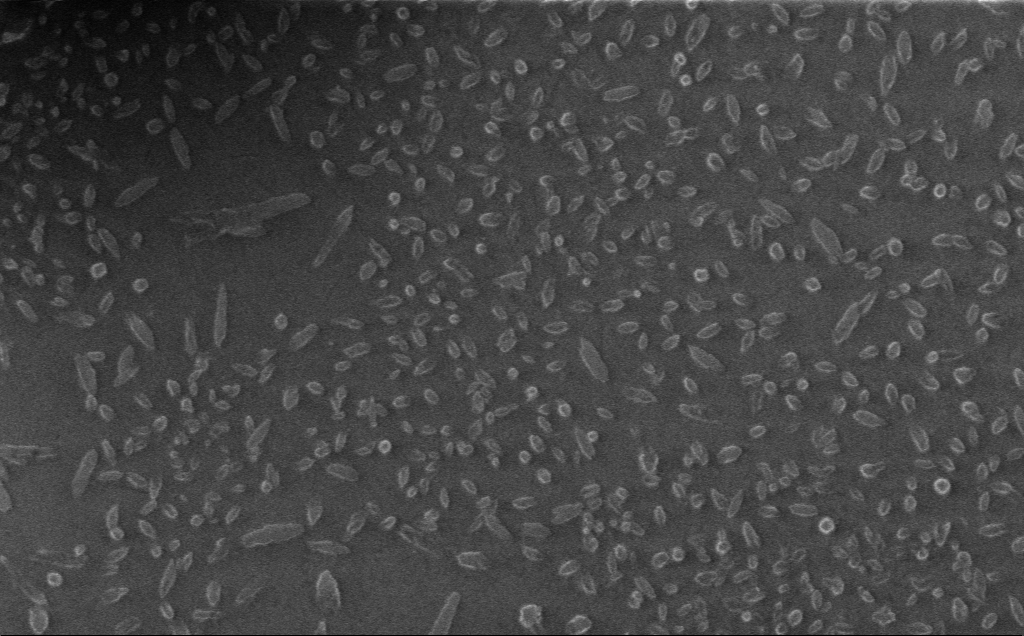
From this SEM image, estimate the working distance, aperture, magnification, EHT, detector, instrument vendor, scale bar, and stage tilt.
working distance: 3 mm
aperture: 30 µm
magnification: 8.98 K X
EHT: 1 kV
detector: InLens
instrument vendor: Zeiss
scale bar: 2000 nm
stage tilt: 0°